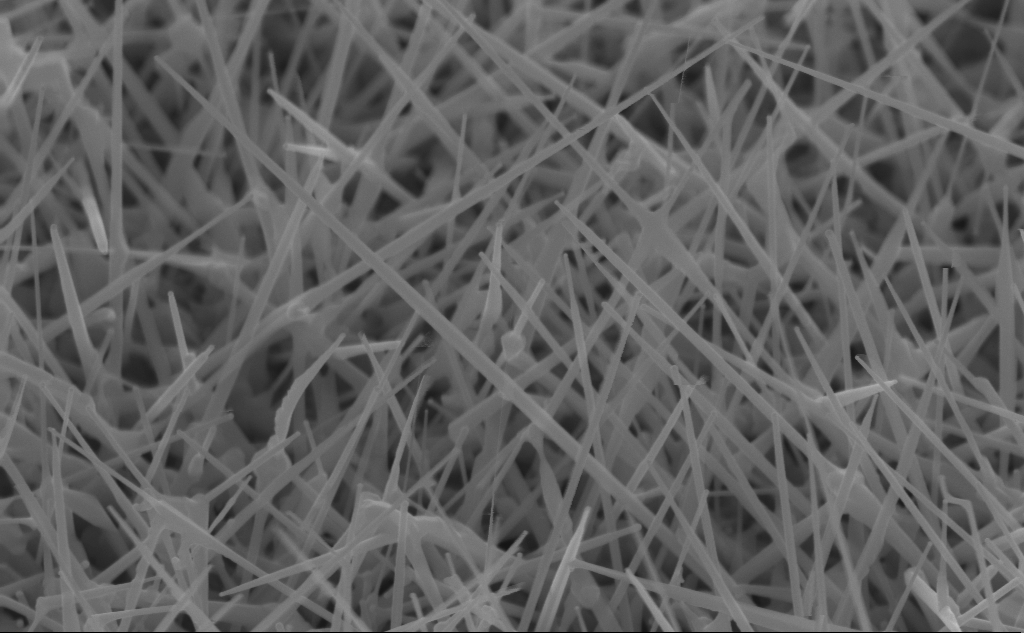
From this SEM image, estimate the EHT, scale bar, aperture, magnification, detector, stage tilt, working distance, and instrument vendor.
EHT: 10 kV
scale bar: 1000 nm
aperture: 30 µm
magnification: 60 K X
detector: InLens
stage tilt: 45°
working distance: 4 mm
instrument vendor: Zeiss